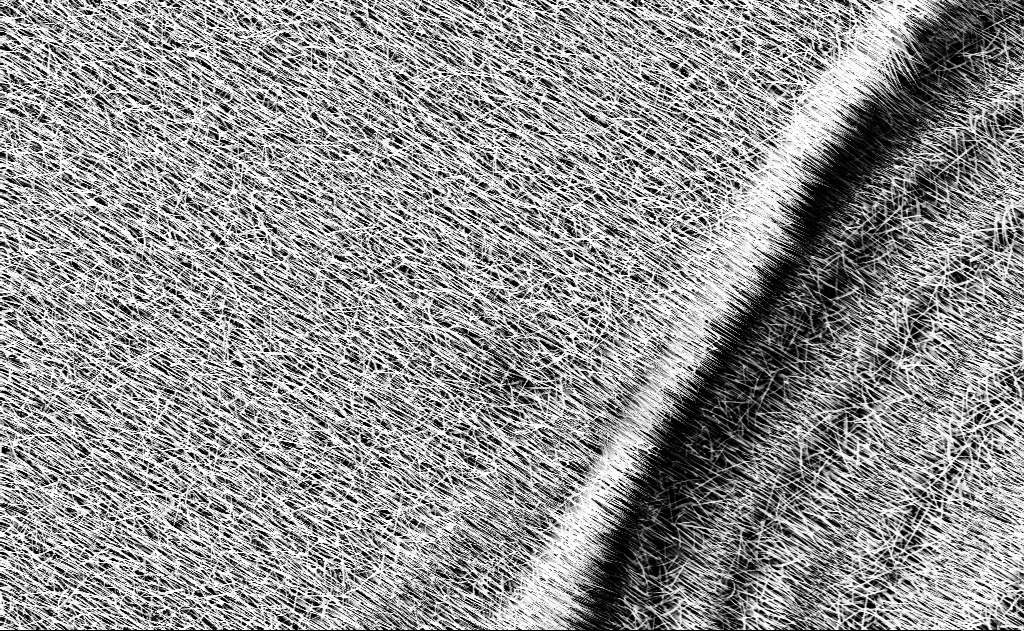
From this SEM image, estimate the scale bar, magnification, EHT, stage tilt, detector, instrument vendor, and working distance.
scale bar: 10000 nm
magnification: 5 K X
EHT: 10 kV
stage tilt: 0°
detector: InLens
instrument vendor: Zeiss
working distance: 13 mm